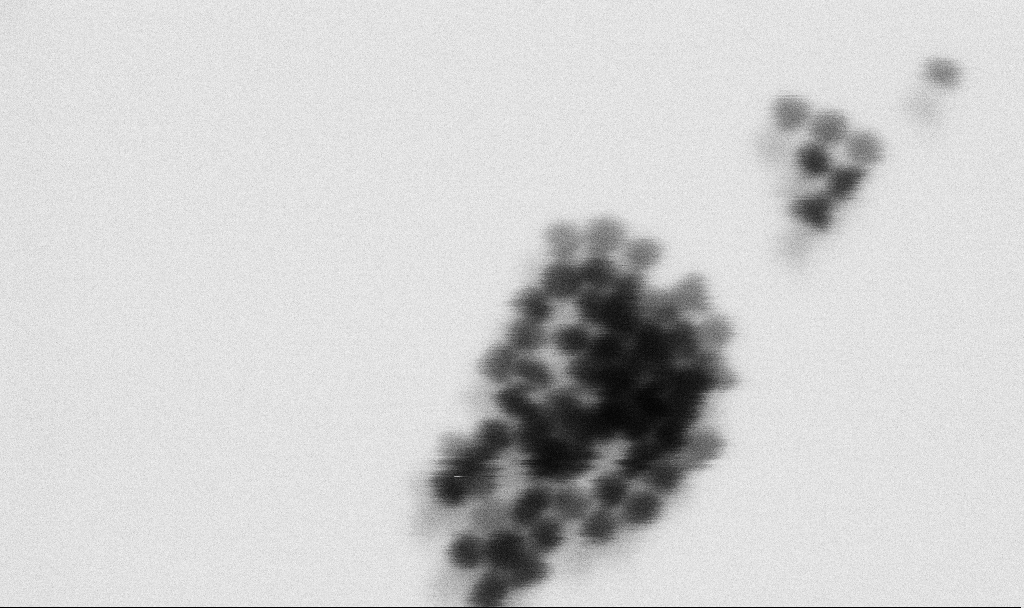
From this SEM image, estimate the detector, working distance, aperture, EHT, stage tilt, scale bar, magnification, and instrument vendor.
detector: SE2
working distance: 5 mm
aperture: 30 µm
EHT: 3 kV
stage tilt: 0°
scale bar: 20 nm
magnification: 736.15 K X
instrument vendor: Zeiss